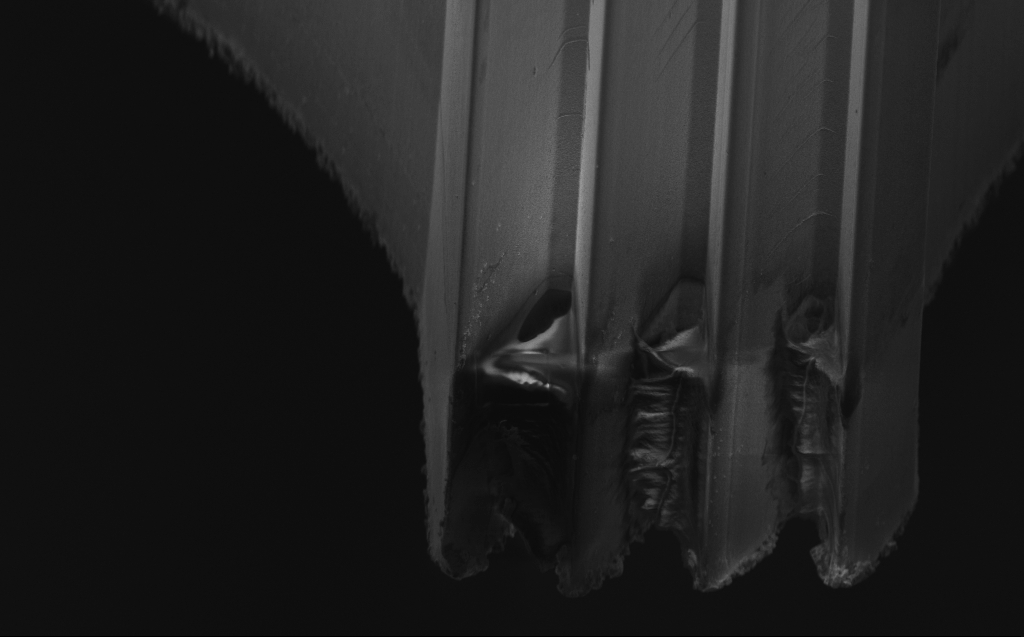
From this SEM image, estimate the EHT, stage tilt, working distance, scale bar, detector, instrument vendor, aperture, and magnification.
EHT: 3 kV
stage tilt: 45°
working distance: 8 mm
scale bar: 10000 nm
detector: InLens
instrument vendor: Zeiss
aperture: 30 µm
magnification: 4.26 K X